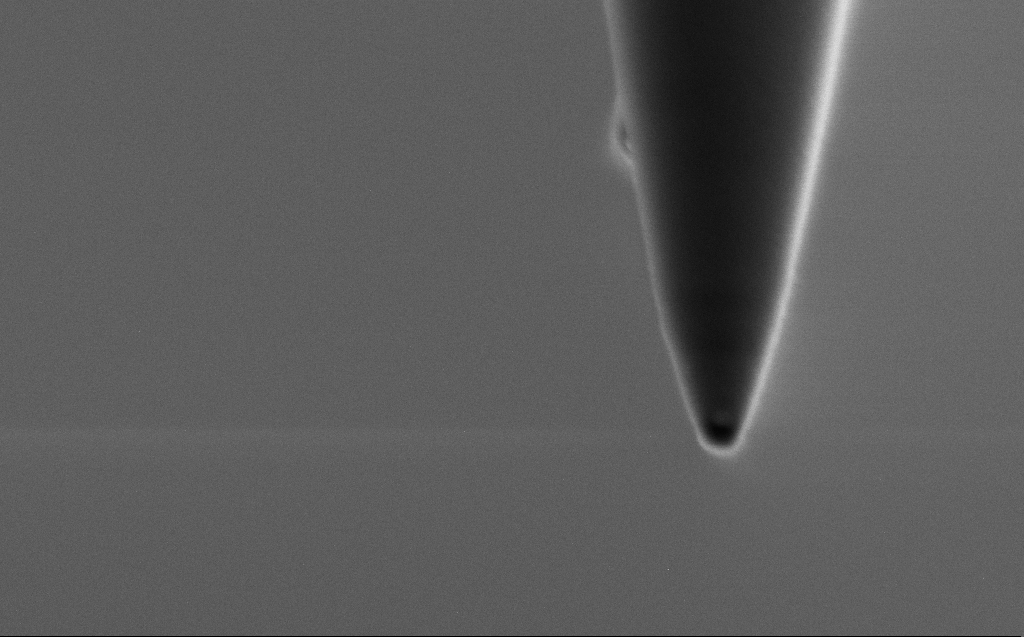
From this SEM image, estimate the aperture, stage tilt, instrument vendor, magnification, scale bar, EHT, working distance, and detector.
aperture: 30 µm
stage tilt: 45.1°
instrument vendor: Zeiss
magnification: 161.01 K X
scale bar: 200 nm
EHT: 0.75 kV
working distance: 3 mm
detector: SE2